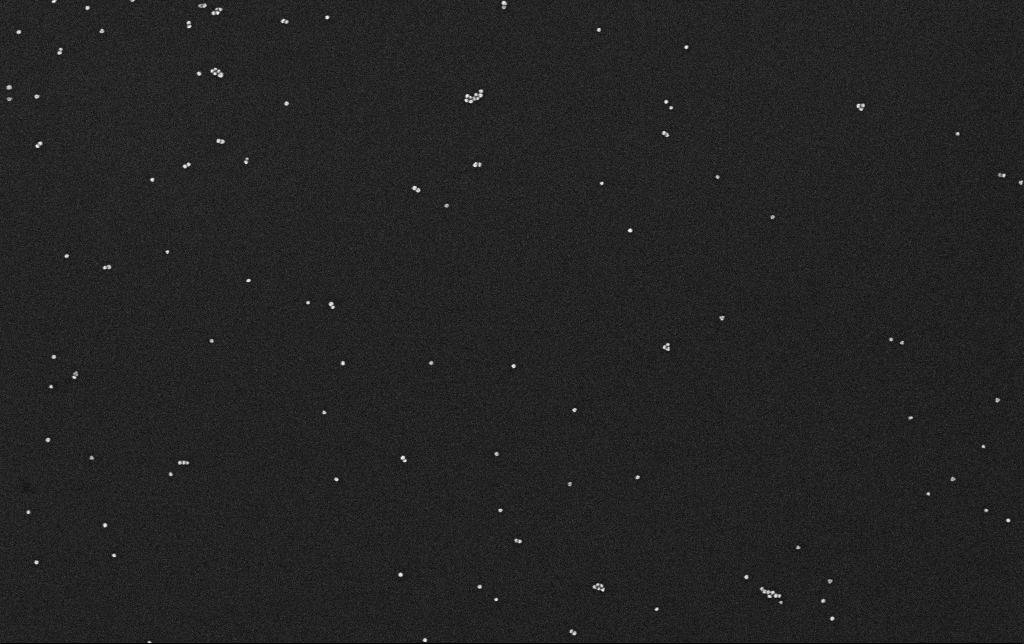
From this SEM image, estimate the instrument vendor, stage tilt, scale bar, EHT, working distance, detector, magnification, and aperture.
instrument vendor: Zeiss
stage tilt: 0°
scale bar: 200 nm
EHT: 10 kV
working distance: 3.1 mm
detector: InLens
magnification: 100 K X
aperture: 30 µm